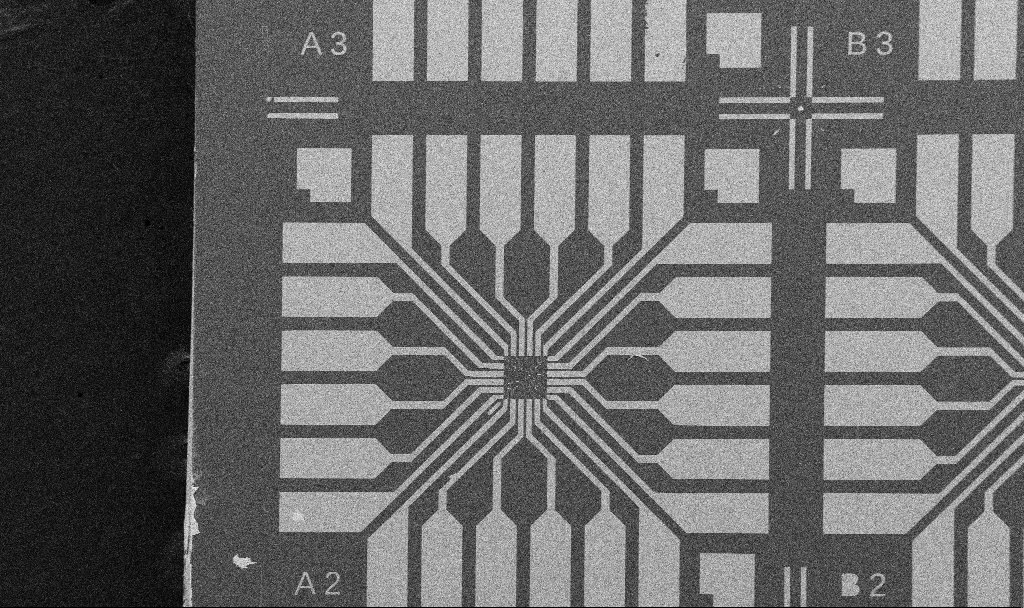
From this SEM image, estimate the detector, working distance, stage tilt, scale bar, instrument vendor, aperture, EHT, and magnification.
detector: SE2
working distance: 10.7 mm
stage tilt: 0°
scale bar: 200000 nm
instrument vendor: Zeiss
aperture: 30 µm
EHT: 10 kV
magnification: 0.1 K X